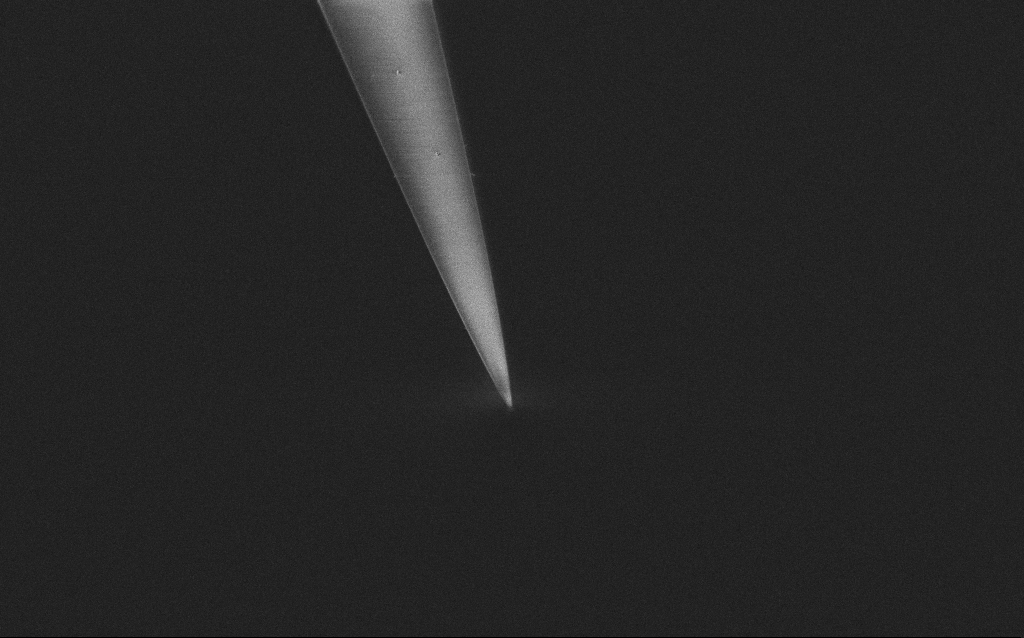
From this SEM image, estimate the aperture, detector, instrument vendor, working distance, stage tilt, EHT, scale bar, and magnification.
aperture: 30 µm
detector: InLens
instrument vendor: Zeiss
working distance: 7 mm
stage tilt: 45°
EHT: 1 kV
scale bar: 2000 nm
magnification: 10 K X